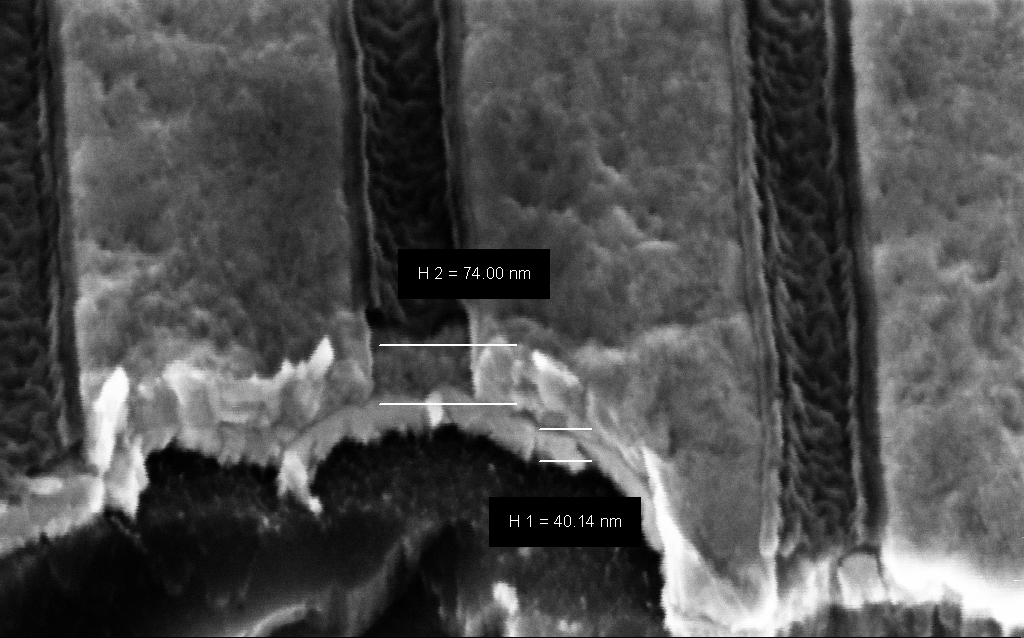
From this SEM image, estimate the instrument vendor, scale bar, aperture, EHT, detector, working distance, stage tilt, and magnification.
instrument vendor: Zeiss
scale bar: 100 nm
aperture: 30 µm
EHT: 3 kV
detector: InLens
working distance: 6 mm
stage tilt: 45°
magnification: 292.75 K X